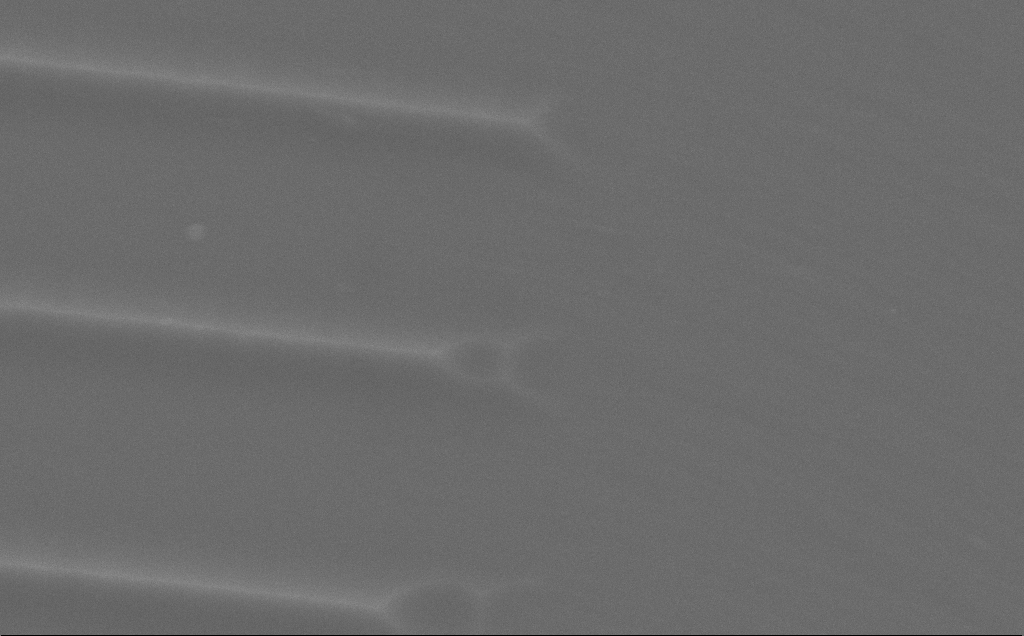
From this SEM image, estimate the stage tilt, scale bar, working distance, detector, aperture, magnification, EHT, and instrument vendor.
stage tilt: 0°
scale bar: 2000 nm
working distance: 11 mm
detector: SE2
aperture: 30 µm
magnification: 14.67 K X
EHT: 10 kV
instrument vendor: Zeiss